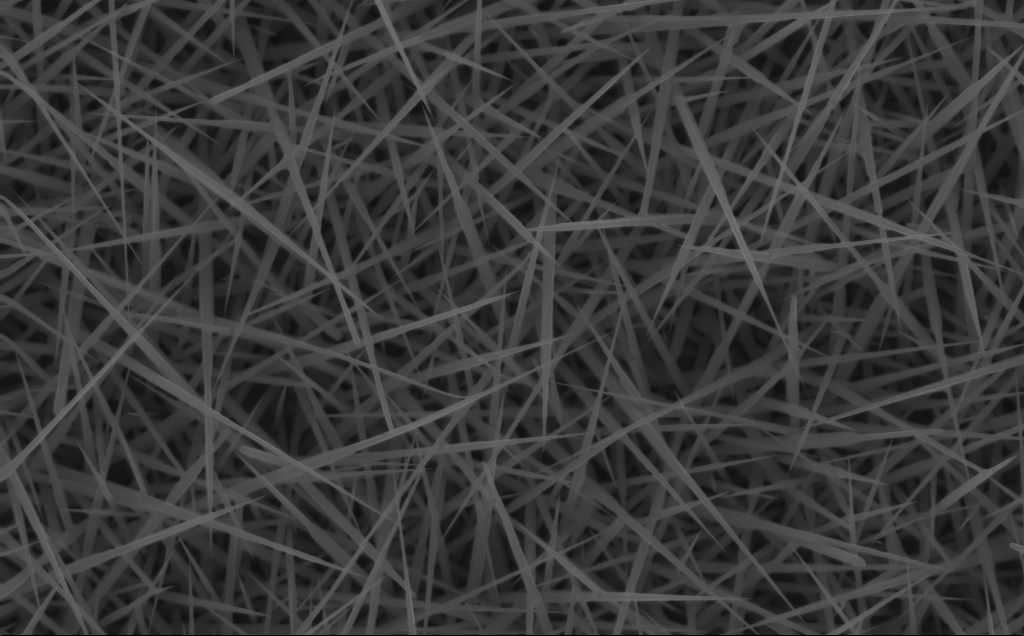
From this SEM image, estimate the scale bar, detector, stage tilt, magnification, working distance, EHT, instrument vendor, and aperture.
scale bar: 1000 nm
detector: InLens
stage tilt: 0°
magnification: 40 K X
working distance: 4 mm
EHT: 10 kV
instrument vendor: Zeiss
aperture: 30 µm